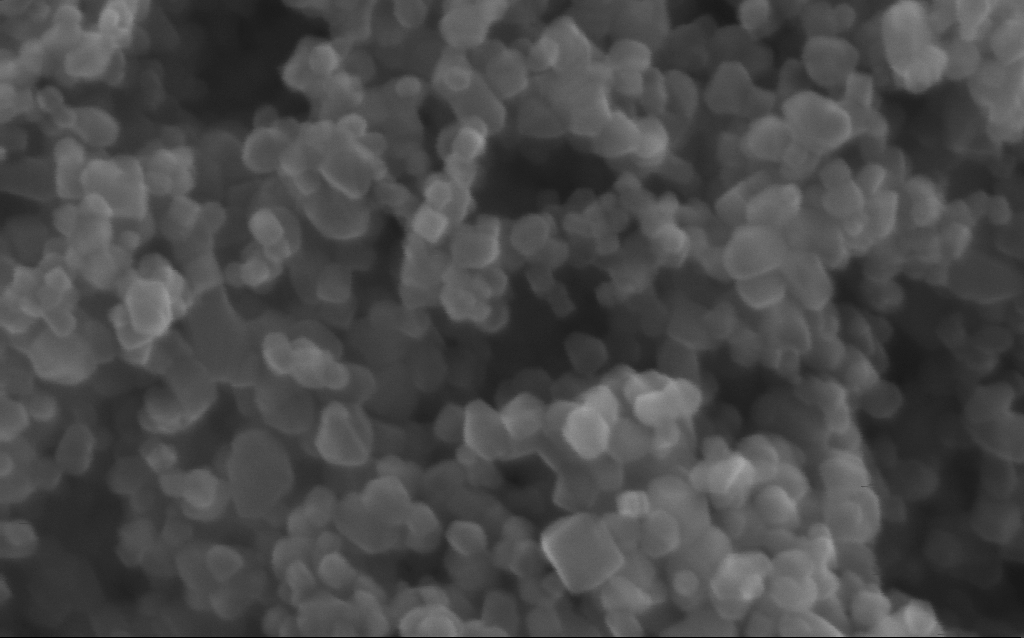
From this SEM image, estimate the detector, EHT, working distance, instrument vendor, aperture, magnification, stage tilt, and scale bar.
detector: InLens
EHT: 10 kV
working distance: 2.7 mm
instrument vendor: Zeiss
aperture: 30 µm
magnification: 600 K X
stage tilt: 0°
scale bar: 100 nm